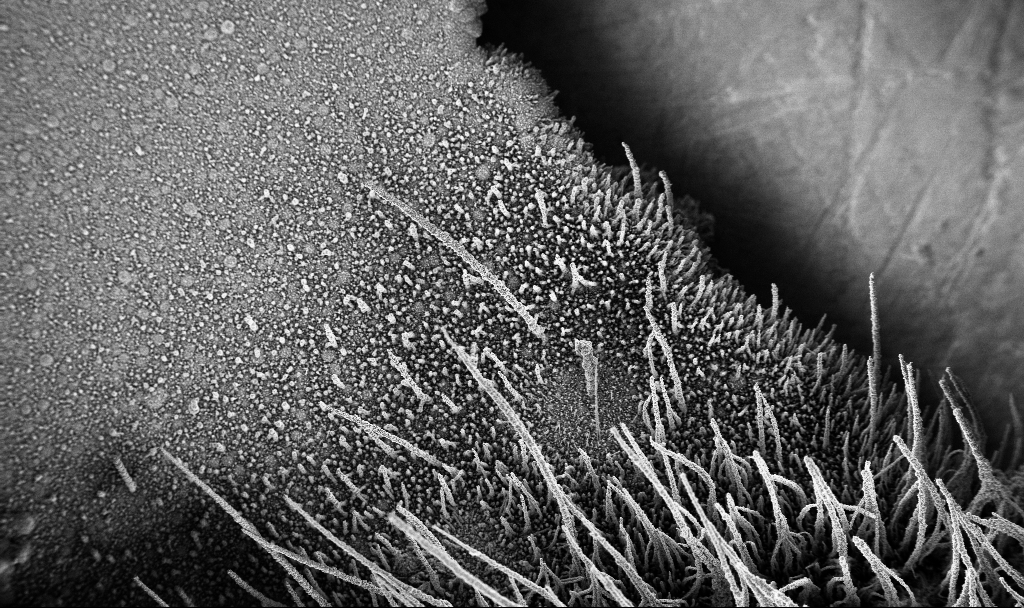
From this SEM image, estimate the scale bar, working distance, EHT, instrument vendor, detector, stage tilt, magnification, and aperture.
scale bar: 100000 nm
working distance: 3.8 mm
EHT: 3 kV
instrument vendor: Zeiss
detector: InLens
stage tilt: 0°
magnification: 0.15 K X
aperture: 30 µm